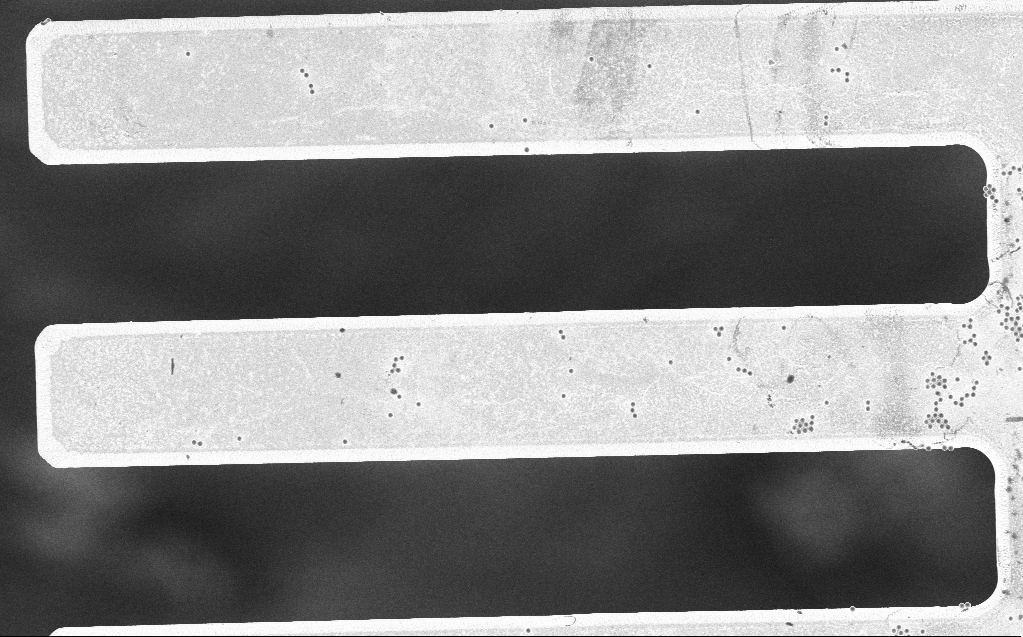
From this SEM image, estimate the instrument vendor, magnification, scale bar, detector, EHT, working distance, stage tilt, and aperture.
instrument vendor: Zeiss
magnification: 2.8 K X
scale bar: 20000 nm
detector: InLens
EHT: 3 kV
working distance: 7 mm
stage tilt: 0°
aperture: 30 µm